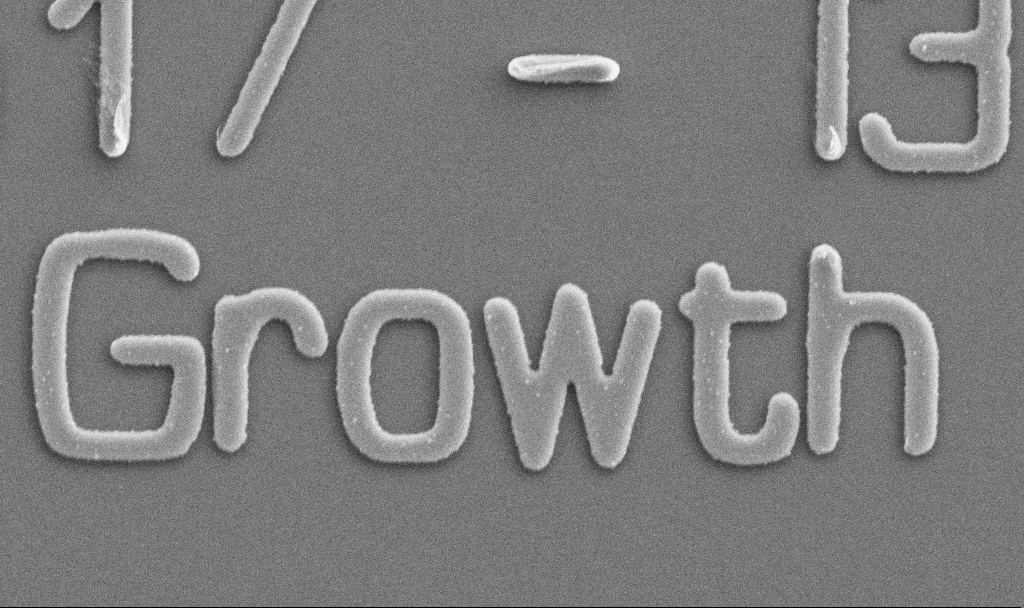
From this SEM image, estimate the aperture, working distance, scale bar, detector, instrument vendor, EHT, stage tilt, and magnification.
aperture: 30 µm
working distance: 10.7 mm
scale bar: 1000 nm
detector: SE2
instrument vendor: Zeiss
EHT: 5 kV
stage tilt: -0°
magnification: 20 K X